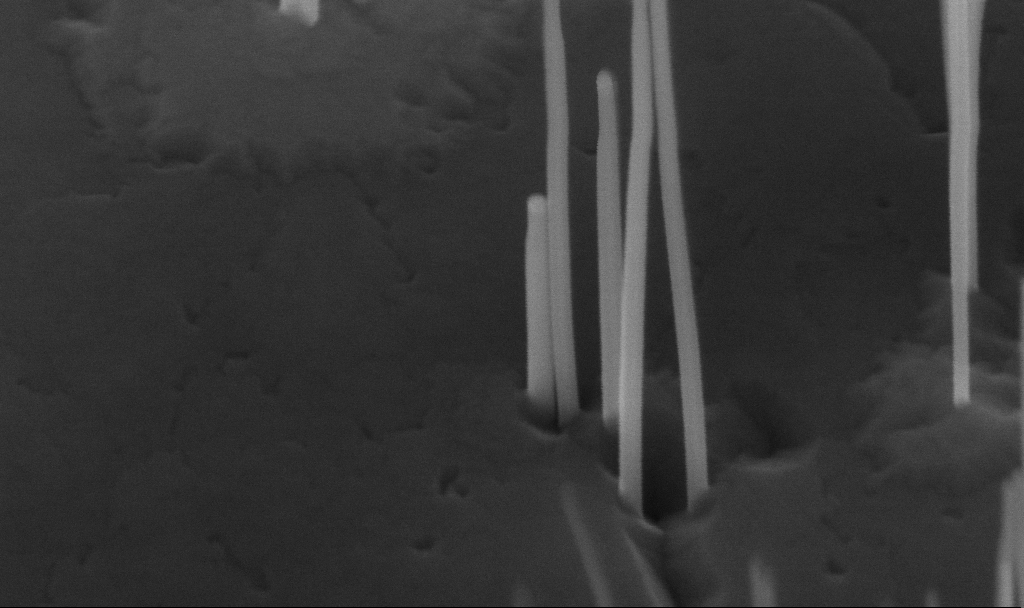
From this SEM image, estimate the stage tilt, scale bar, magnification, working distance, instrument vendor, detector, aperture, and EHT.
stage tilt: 45°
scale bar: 200 nm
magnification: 280 K X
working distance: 5.6 mm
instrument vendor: Zeiss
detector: InLens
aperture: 30 µm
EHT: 10 kV